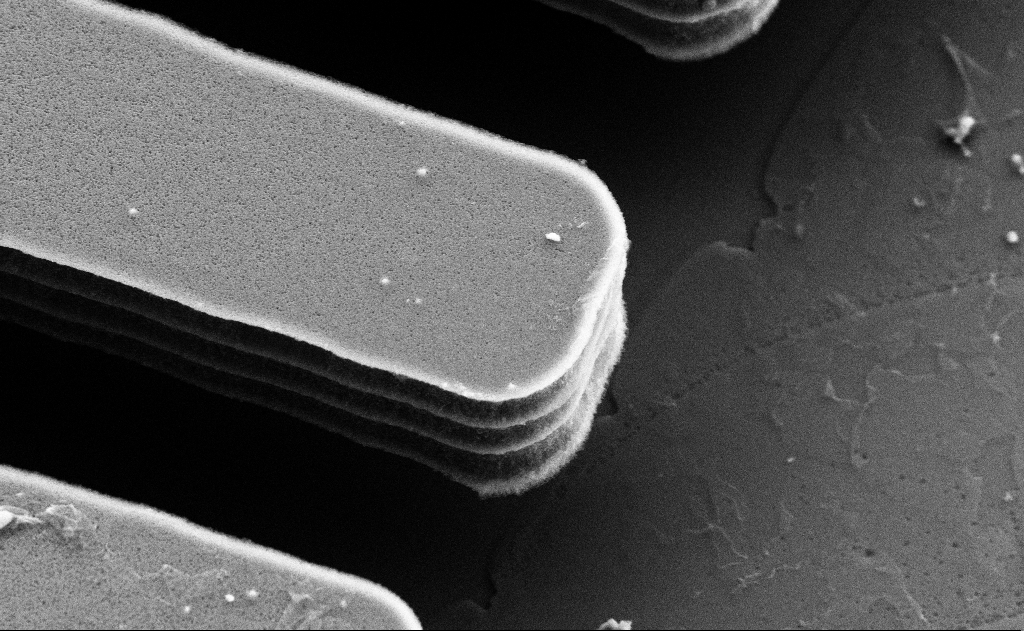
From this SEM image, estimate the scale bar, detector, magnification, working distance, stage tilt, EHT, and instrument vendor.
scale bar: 1000 nm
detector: SE2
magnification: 19.89 K X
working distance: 8 mm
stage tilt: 31.8°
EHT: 5 kV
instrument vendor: Zeiss